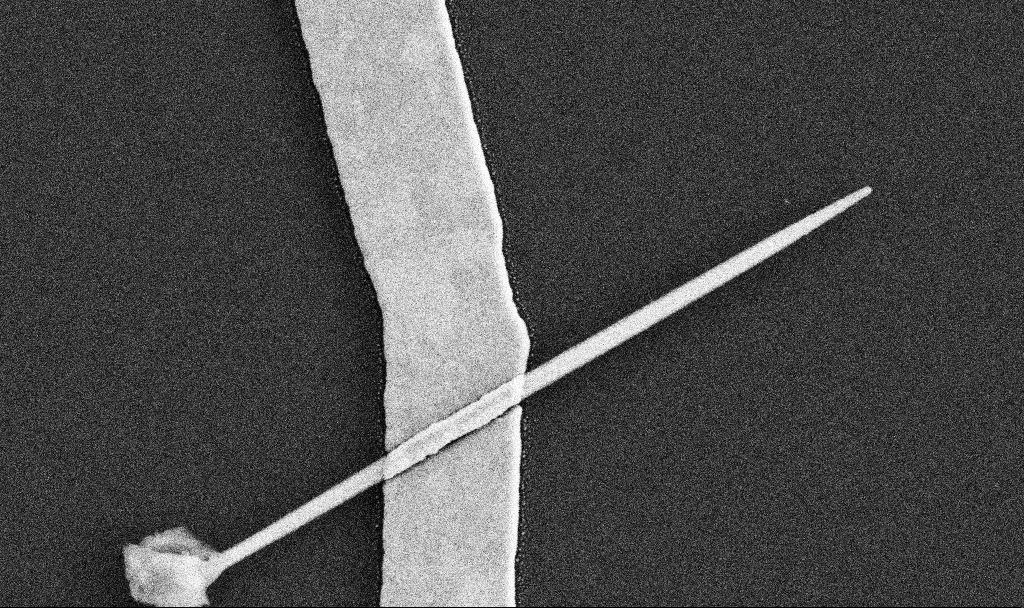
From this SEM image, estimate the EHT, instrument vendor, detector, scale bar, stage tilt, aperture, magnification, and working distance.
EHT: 5 kV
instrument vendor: Zeiss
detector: SE2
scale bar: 1000 nm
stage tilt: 0°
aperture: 30 µm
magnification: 56.96 K X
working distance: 7.6 mm